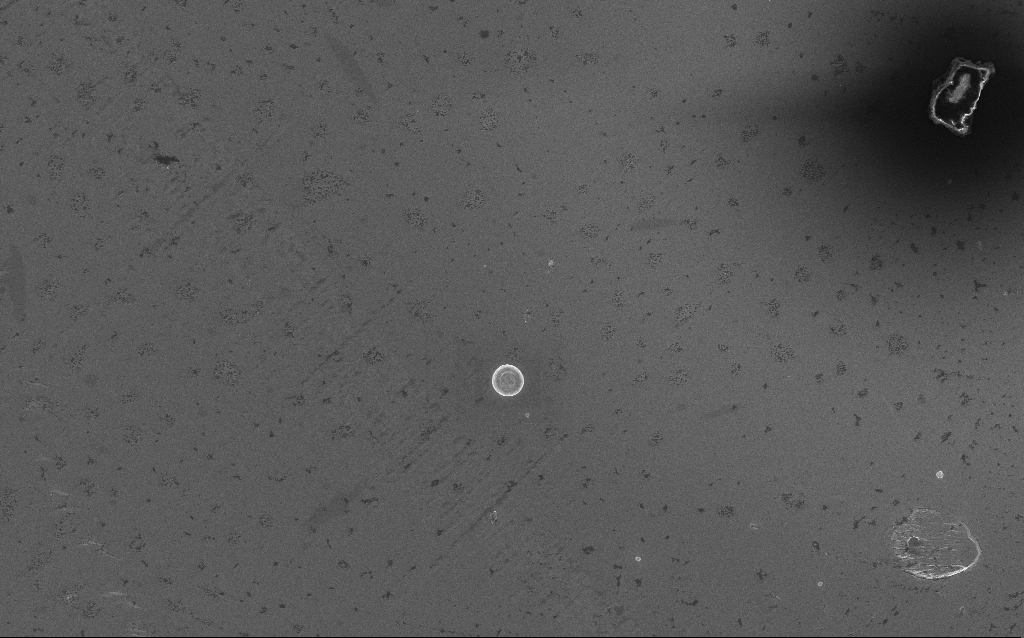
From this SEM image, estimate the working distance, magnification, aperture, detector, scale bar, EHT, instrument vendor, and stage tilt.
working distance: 5 mm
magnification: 3.11 K X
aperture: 30 µm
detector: InLens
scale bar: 10000 nm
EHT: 5 kV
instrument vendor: Zeiss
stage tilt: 0°